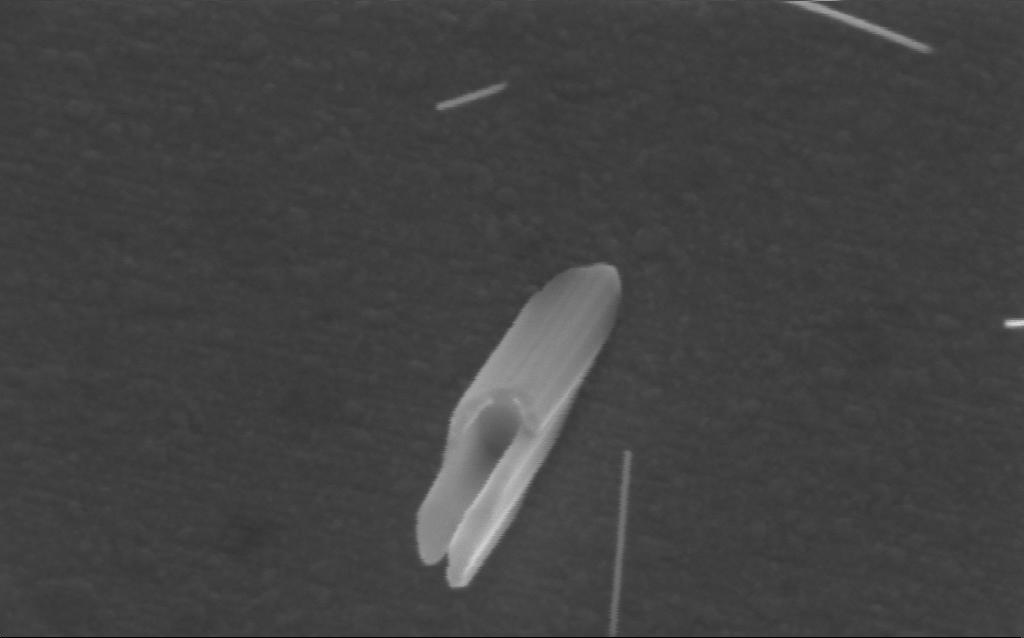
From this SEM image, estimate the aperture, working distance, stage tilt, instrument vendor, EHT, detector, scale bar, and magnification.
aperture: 30 µm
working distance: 3 mm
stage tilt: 23.6°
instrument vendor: Zeiss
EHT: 5 kV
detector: InLens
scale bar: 200 nm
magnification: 316.13 K X